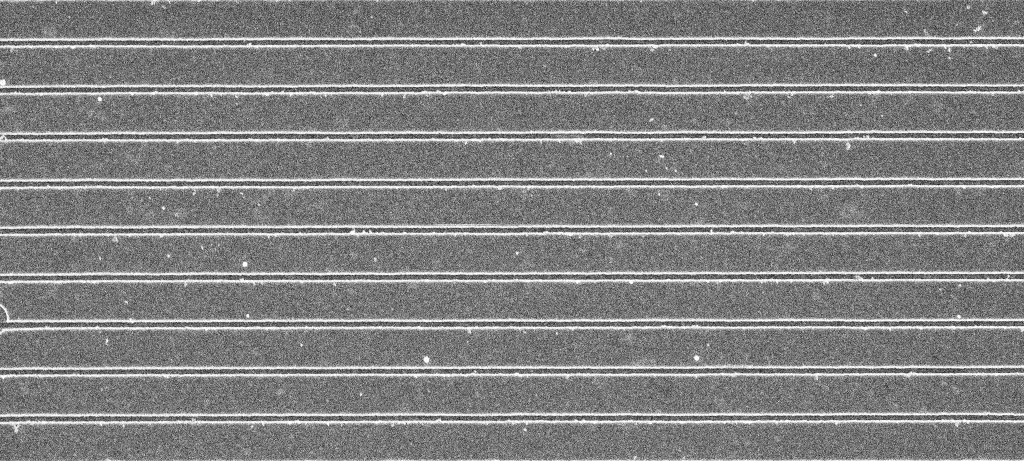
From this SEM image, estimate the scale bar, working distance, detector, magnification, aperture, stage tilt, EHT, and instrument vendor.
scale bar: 2000 nm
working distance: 5.4 mm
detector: InLens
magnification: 19.28 K X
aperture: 30 µm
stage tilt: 0°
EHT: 5 kV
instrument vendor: Zeiss